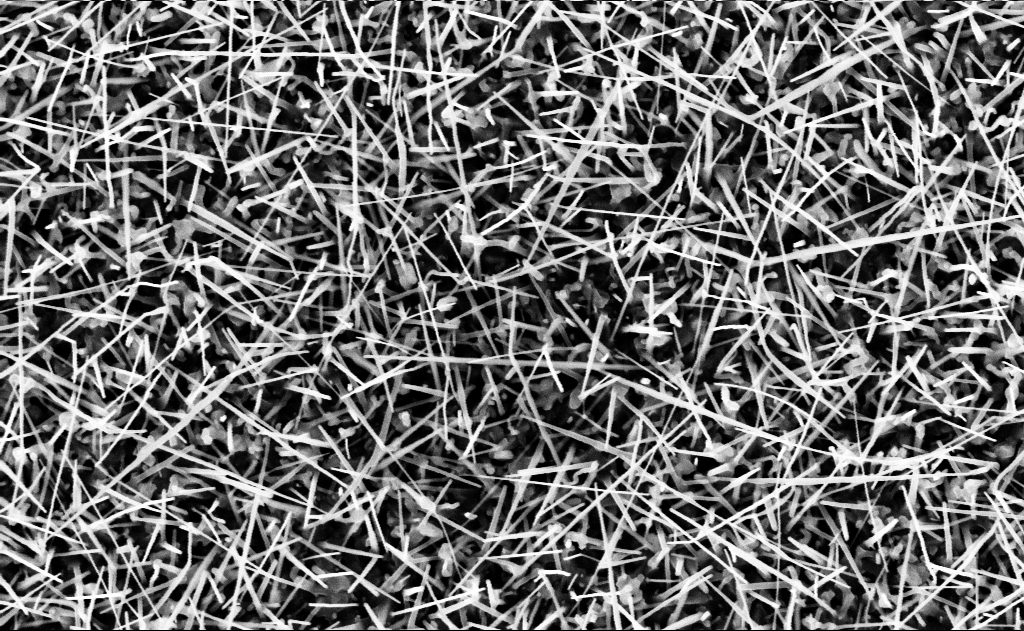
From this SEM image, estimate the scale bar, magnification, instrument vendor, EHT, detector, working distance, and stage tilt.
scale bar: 1000 nm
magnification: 40 K X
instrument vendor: Zeiss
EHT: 10 kV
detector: InLens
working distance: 15 mm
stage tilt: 0°